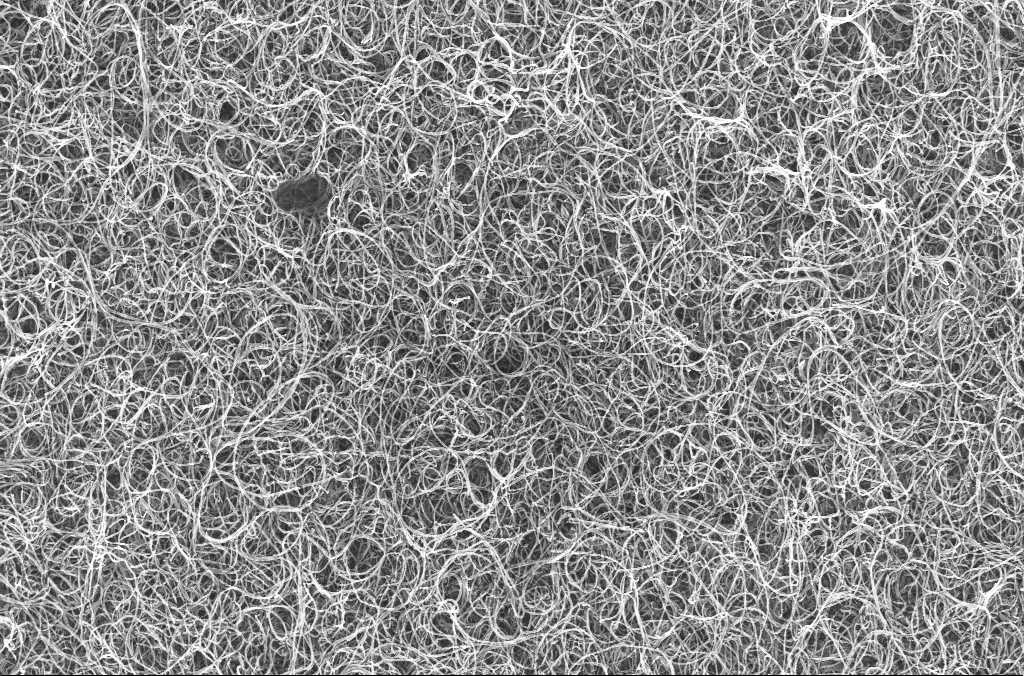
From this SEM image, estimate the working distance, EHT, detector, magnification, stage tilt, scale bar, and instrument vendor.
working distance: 4.5 mm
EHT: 20 kV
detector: InLens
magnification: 20 K X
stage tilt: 0°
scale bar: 1000 nm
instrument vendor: Zeiss